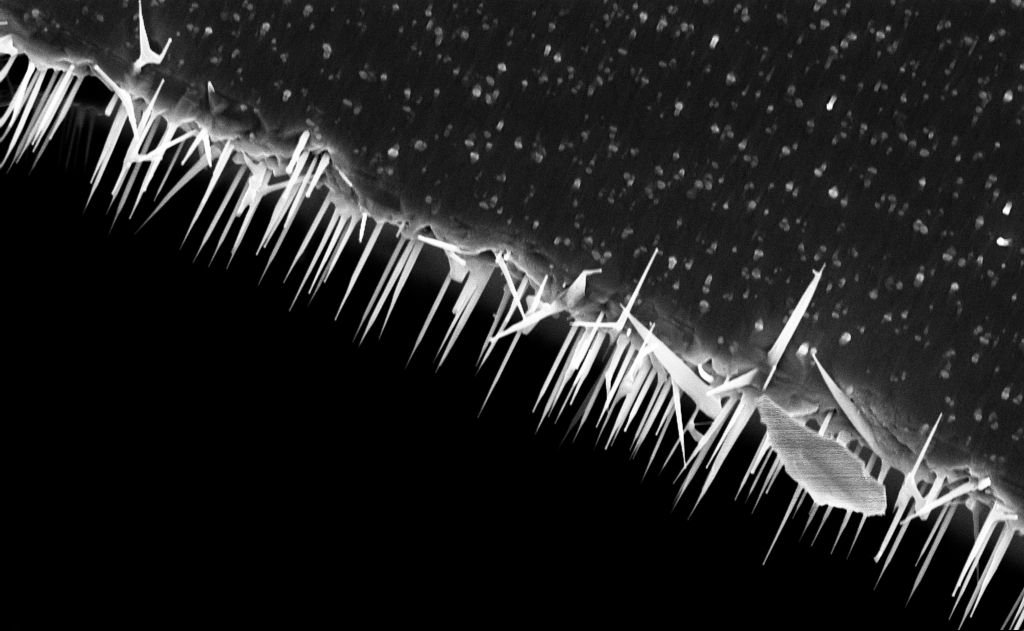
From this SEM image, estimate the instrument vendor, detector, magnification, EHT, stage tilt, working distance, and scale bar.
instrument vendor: Zeiss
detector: InLens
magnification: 20 K X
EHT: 10 kV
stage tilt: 0°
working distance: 9 mm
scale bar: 2000 nm